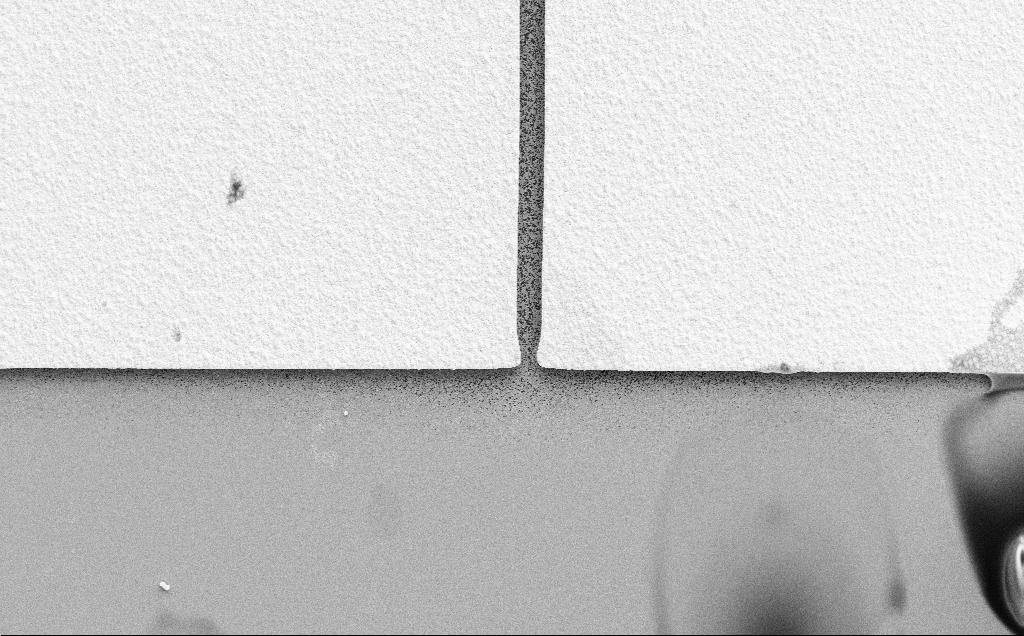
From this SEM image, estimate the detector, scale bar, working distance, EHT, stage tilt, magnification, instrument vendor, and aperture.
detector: SE2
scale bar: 20000 nm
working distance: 17 mm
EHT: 10 kV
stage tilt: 0°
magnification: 0.969 K X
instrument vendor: Zeiss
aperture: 30 µm